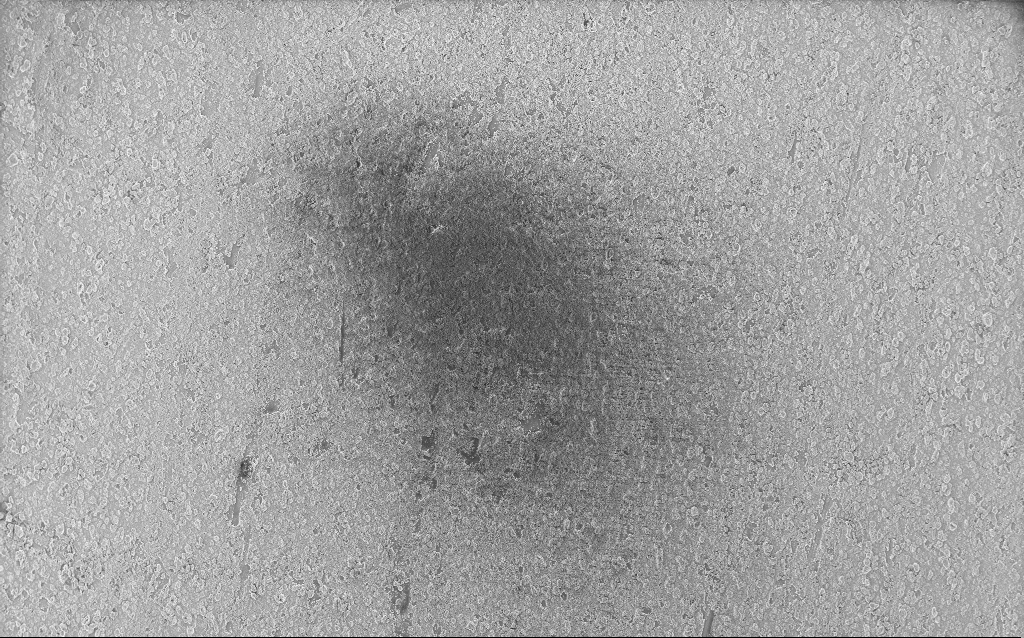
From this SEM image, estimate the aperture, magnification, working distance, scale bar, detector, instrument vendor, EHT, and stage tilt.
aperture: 30 µm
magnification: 0.235 K X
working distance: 2.5 mm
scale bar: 200000 nm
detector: InLens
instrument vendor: Zeiss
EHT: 5 kV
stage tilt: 0°